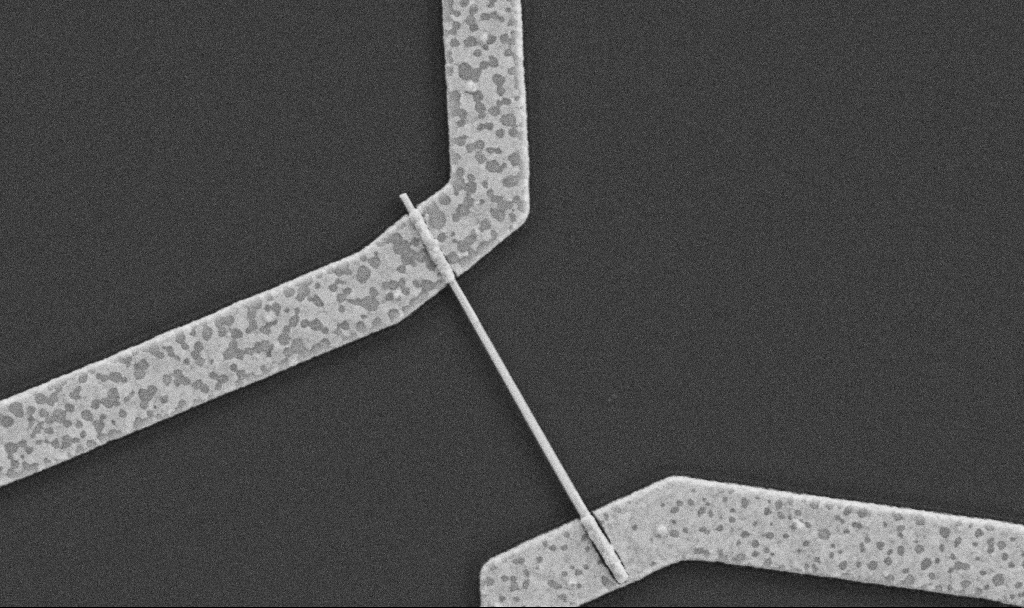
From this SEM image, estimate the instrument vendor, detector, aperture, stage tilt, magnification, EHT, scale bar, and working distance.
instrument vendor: Zeiss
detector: SE2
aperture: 30 µm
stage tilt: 0°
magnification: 30 K X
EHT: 5 kV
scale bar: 1000 nm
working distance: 8.7 mm